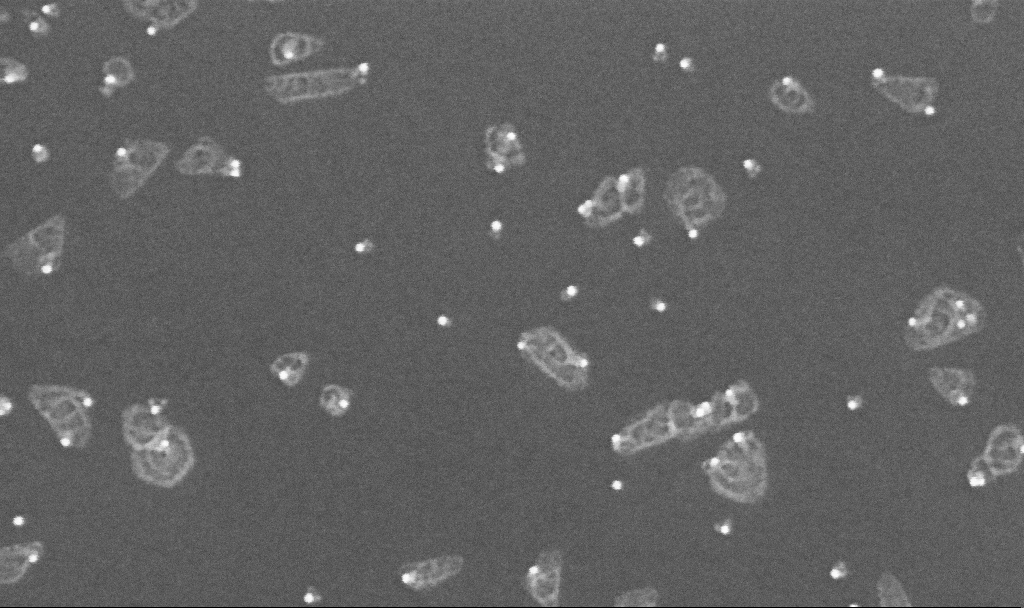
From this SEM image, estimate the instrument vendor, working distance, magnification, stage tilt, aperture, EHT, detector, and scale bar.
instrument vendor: Zeiss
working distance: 3.3 mm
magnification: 350 K X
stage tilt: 0°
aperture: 30 µm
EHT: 10 kV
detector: InLens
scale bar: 100 nm